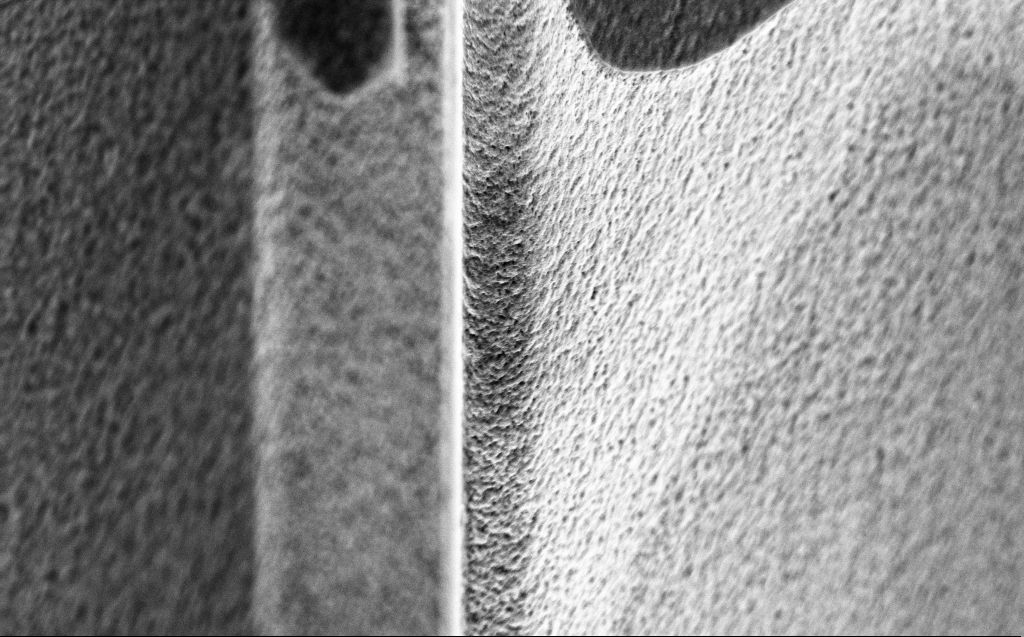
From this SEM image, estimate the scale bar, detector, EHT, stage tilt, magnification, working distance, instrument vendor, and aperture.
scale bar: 2000 nm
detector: InLens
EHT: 5 kV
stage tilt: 45°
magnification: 25.61 K X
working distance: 5 mm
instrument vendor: Zeiss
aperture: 30 µm